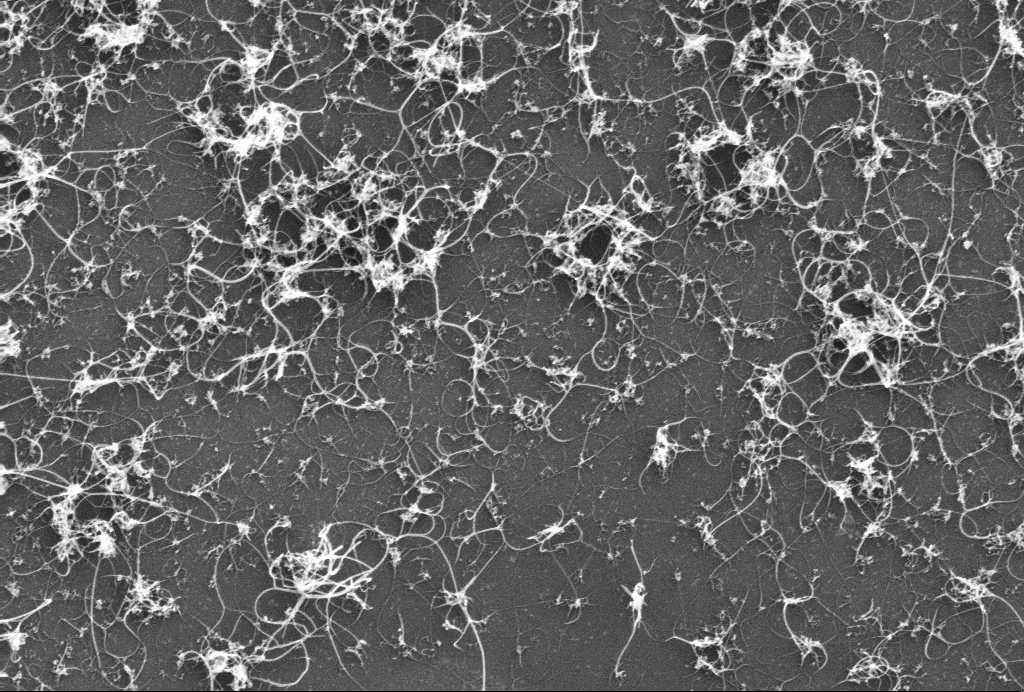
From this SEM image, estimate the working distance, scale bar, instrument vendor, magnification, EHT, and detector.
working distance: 3.1 mm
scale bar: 200 nm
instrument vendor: Zeiss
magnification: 90.32 K X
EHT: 10 kV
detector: InLens